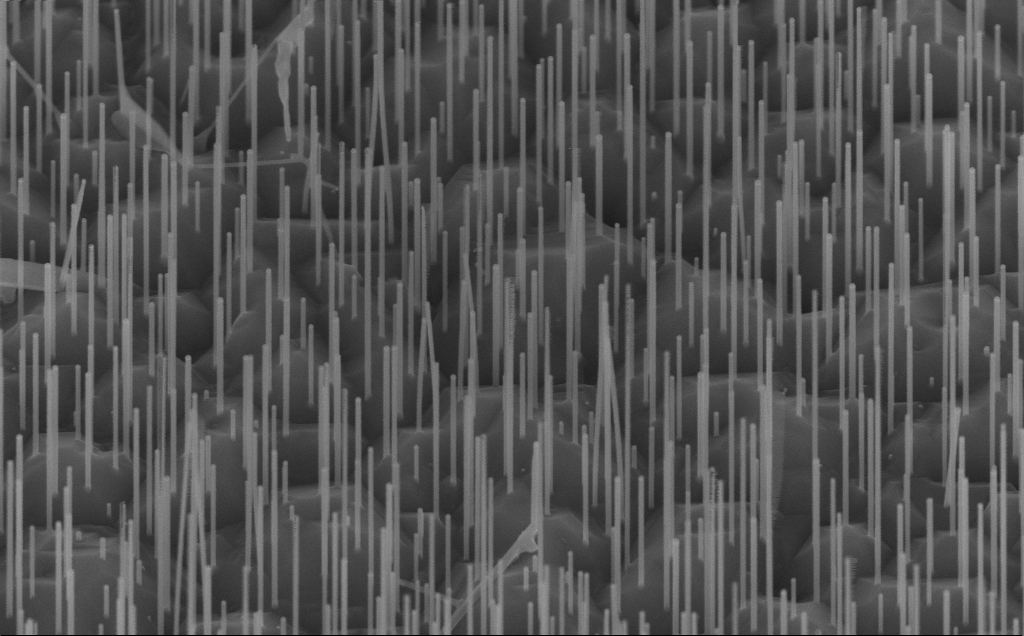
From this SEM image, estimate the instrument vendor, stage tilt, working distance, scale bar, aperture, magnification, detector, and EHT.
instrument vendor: Zeiss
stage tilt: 45°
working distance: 8 mm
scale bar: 1000 nm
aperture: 30 µm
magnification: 65.99 K X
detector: InLens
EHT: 10 kV